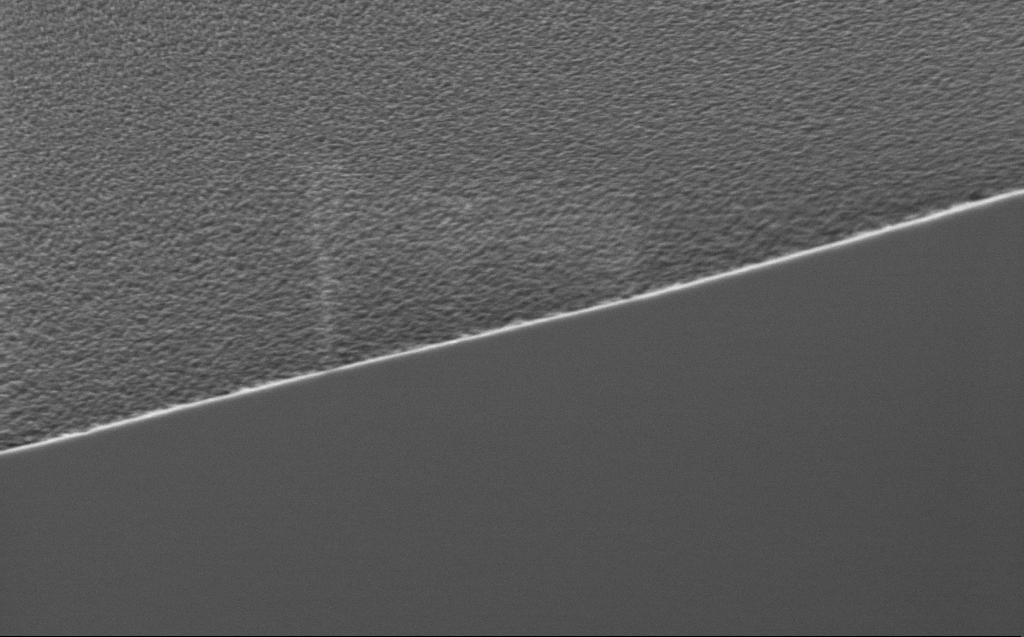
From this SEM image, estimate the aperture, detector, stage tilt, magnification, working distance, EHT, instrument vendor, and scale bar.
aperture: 20 µm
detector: InLens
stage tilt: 44.9°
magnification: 26.93 K X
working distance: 6 mm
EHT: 1.5 kV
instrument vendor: Zeiss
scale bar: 2000 nm